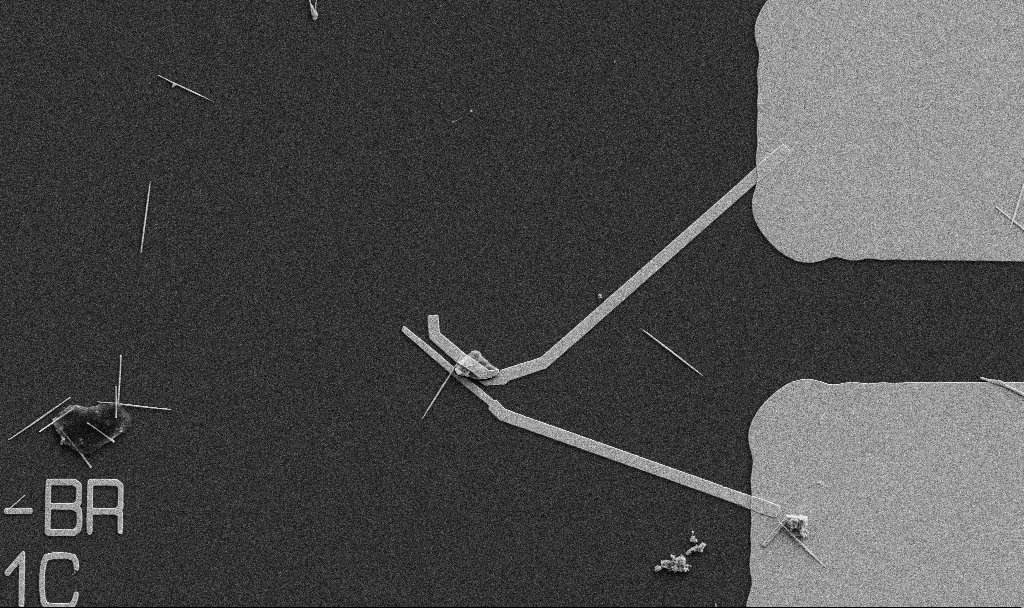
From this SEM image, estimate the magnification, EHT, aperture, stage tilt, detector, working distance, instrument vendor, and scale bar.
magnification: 5 K X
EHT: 5 kV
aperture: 30 µm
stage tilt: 0°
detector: SE2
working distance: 10.7 mm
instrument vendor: Zeiss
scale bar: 10000 nm